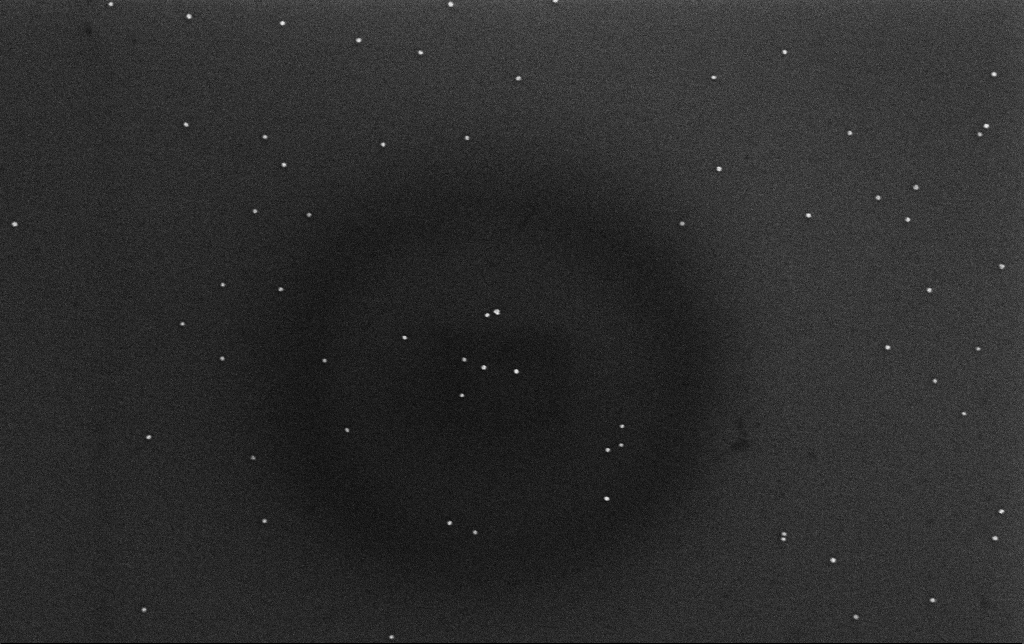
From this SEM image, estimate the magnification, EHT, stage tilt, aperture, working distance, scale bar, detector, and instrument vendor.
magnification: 100 K X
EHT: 10 kV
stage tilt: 0°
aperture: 30 µm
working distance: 3.2 mm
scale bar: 200 nm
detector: InLens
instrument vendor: Zeiss